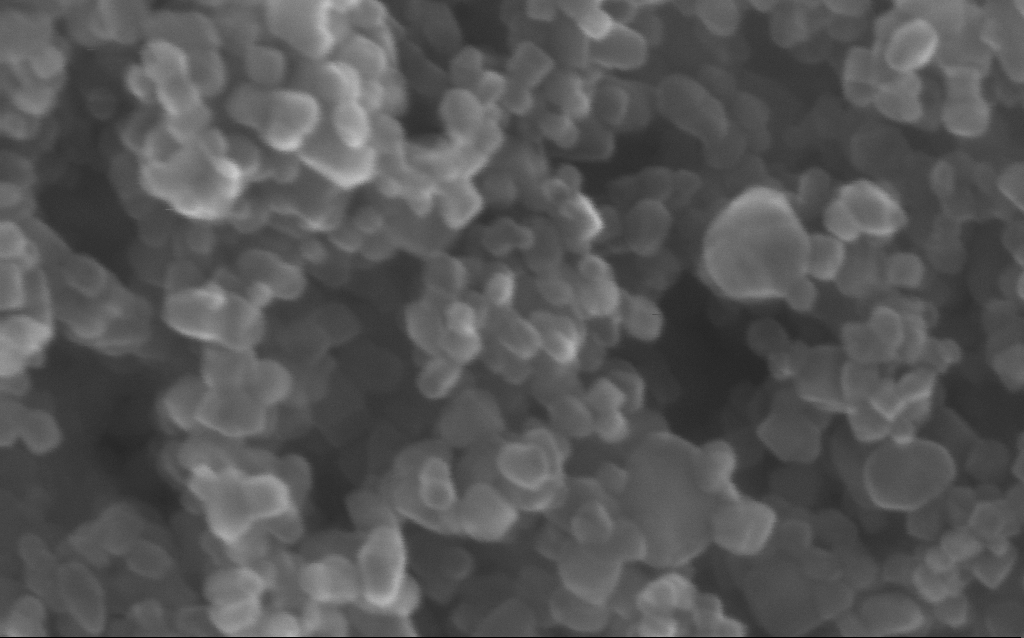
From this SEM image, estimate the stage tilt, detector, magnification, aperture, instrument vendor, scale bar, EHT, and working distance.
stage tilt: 0°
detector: InLens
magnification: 716 K X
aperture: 30 µm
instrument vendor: Zeiss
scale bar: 100 nm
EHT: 15 kV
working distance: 3 mm